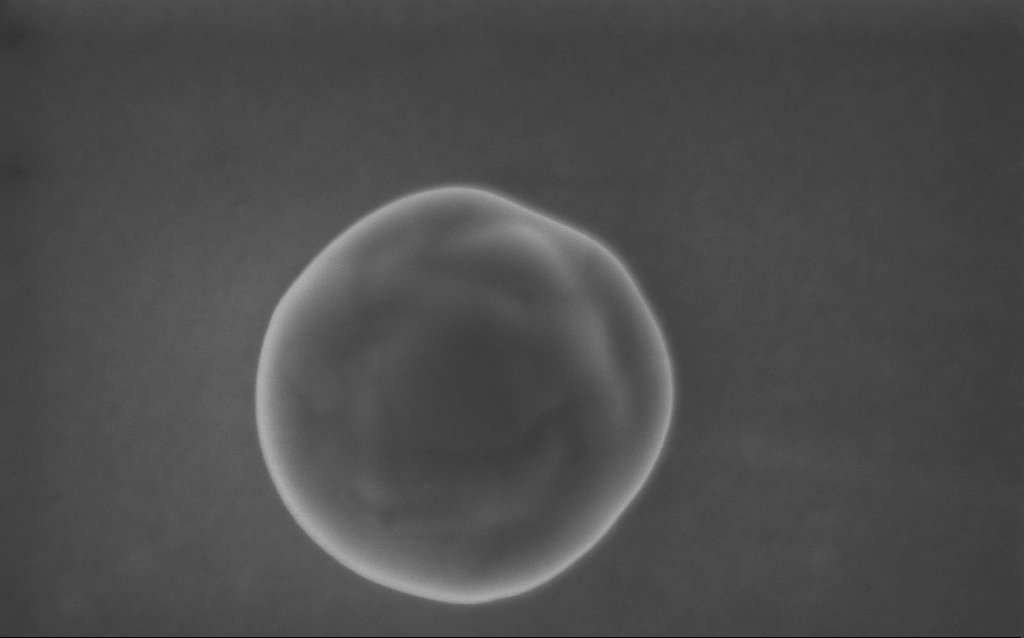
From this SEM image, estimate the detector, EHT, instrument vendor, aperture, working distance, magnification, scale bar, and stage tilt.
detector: InLens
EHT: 5 kV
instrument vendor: Zeiss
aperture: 30 µm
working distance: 4 mm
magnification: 143.12 K X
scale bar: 200 nm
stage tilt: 0°